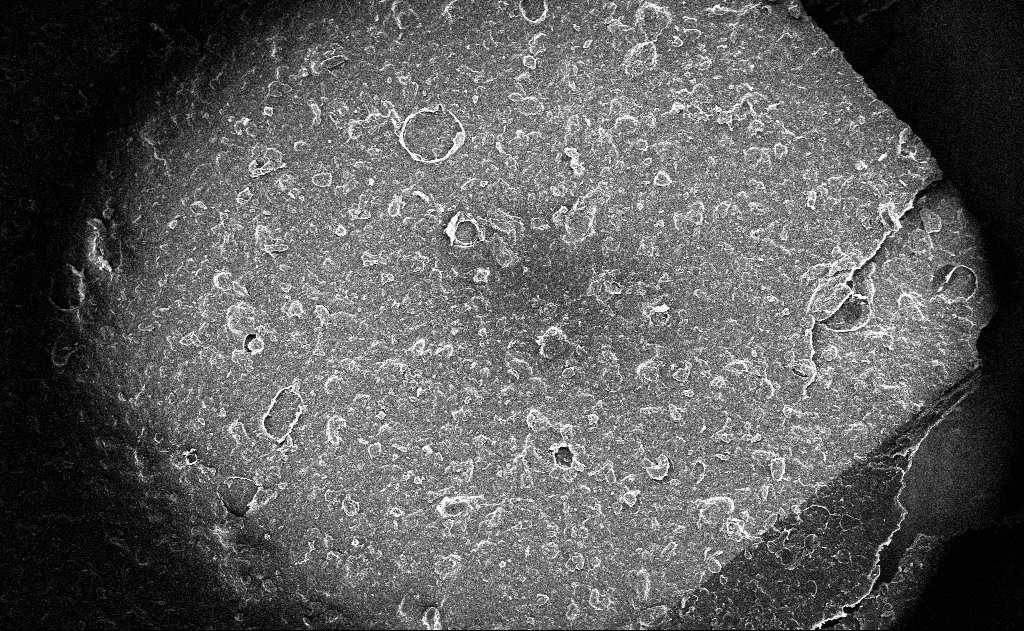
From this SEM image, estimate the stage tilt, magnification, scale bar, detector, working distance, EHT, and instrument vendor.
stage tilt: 0°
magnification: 0.119 K X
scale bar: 200000 nm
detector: InLens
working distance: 3 mm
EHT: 10 kV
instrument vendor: Zeiss